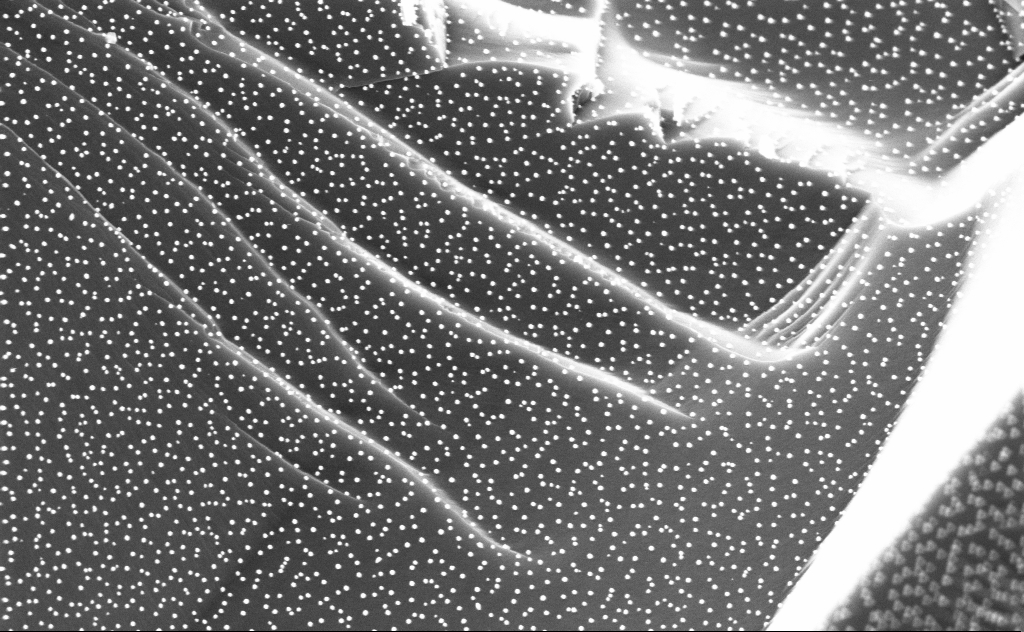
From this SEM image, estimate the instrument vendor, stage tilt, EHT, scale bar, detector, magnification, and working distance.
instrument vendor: Zeiss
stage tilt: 0°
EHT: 3 kV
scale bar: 200 nm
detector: InLens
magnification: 80 K X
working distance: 5 mm